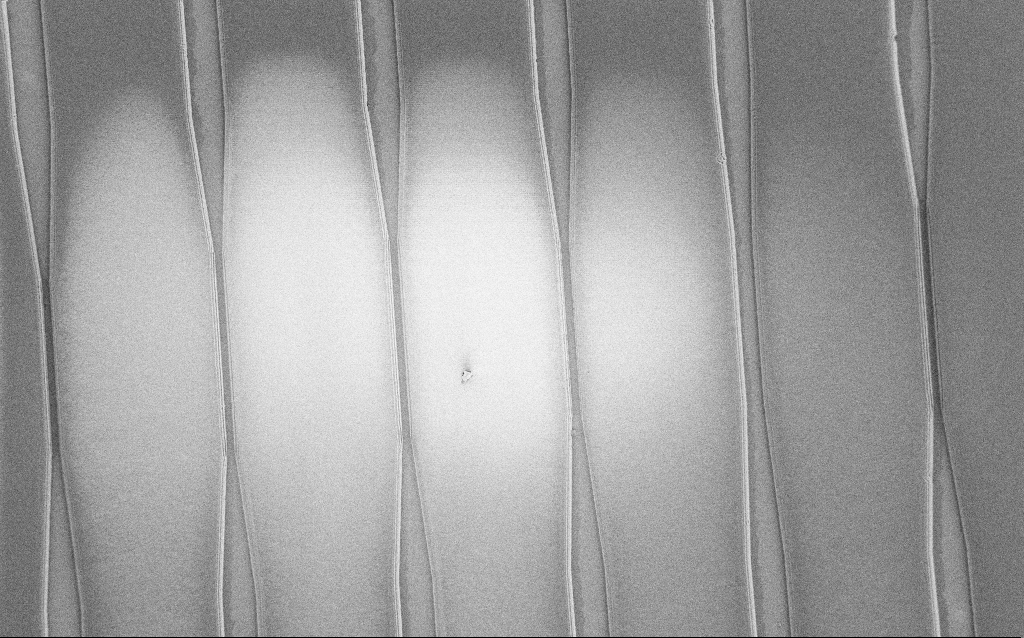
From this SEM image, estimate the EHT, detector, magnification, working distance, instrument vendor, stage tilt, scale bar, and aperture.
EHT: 1 kV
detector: SE2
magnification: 0.604 K X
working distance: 6 mm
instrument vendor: Zeiss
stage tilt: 0°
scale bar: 100000 nm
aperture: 30 µm